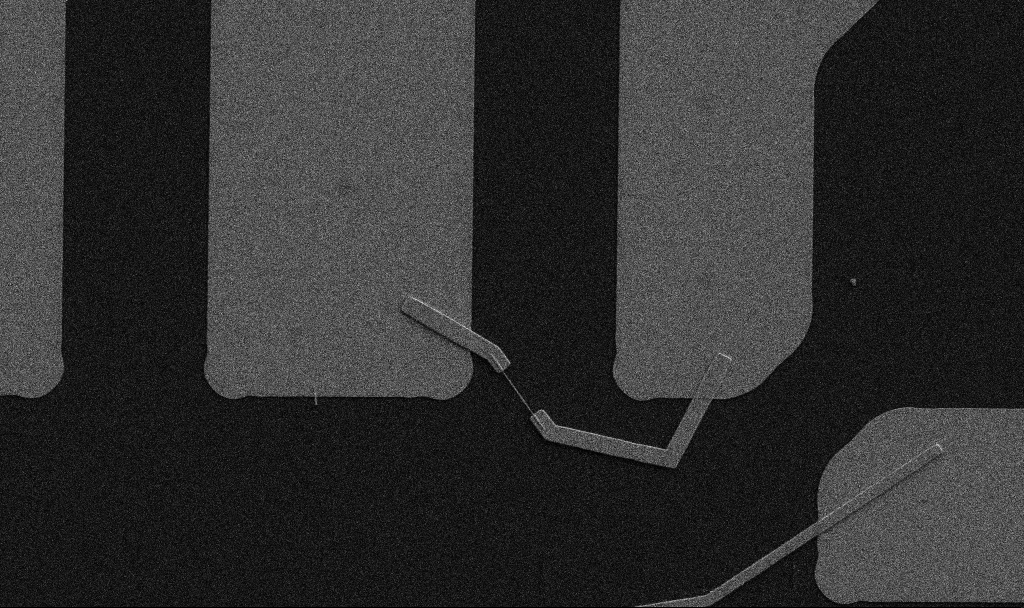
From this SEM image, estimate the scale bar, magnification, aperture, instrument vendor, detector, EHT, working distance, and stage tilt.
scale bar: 10000 nm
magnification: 5 K X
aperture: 30 µm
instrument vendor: Zeiss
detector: SE2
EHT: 5 kV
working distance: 10.7 mm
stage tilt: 0°